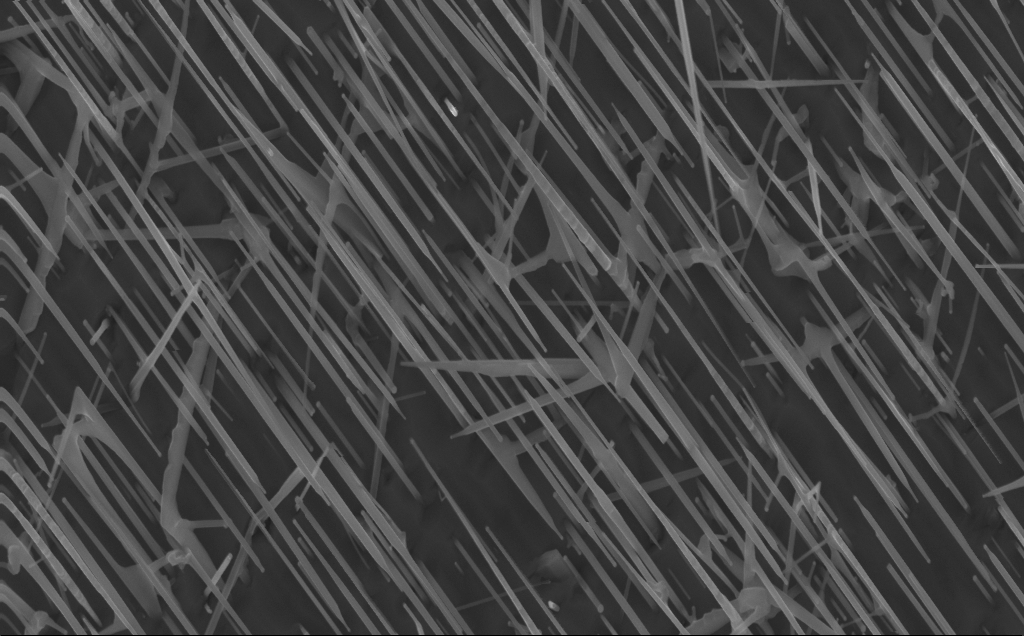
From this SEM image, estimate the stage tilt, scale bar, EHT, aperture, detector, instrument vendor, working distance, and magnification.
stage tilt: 0°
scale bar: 1000 nm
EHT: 10 kV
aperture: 30 µm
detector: InLens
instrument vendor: Zeiss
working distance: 4 mm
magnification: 40 K X